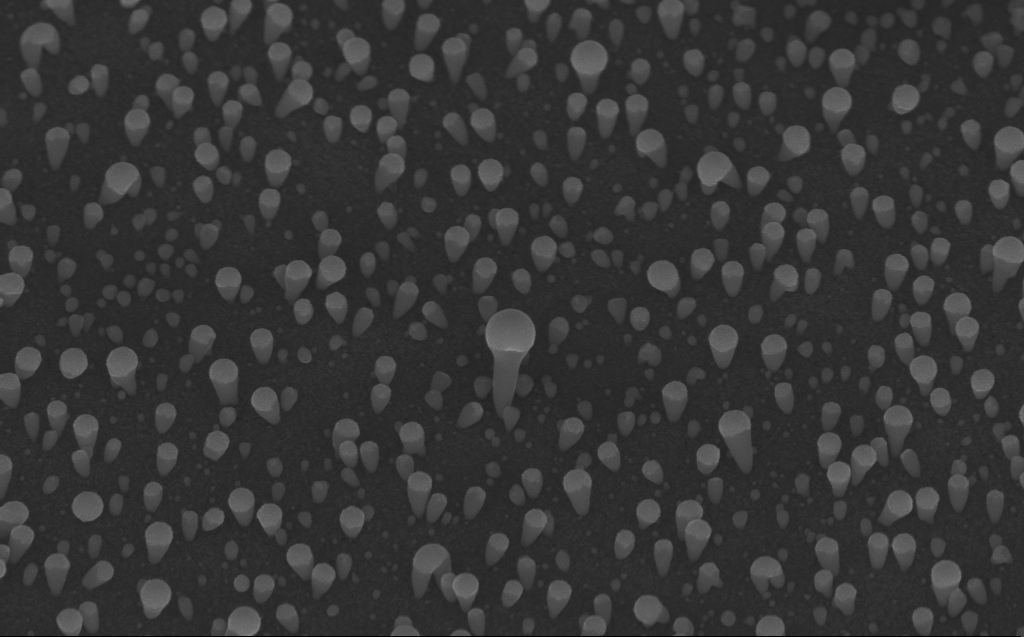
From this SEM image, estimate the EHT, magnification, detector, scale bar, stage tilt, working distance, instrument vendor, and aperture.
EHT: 10 kV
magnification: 50 K X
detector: InLens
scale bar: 1000 nm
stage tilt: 45°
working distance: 7 mm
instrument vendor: Zeiss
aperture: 30 µm